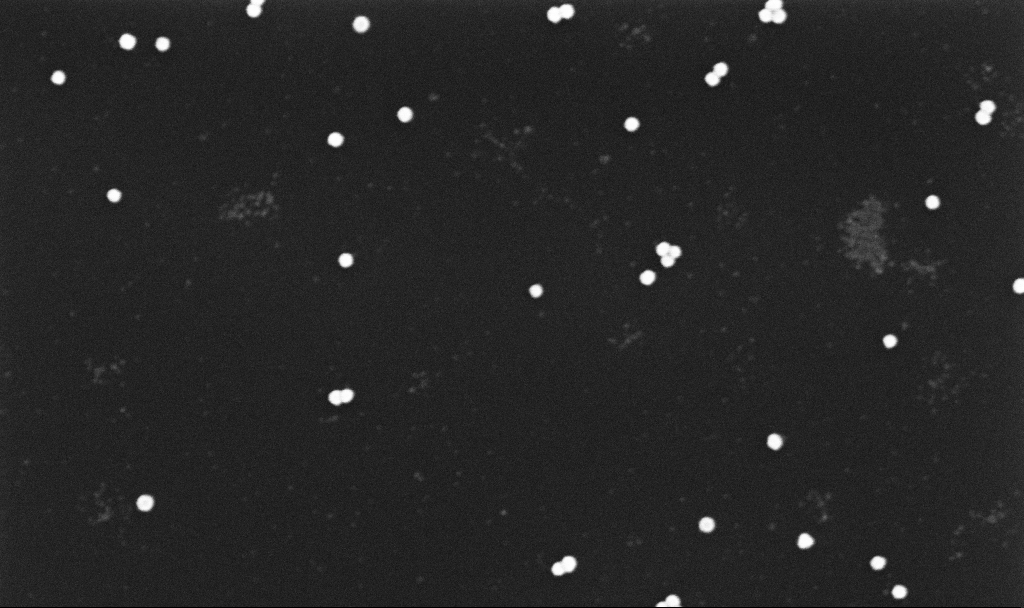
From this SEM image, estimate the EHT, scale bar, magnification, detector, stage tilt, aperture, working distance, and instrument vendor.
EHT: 10 kV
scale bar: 1000 nm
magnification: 70 K X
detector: InLens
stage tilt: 0°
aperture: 30 µm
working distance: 3.3 mm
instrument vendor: Zeiss